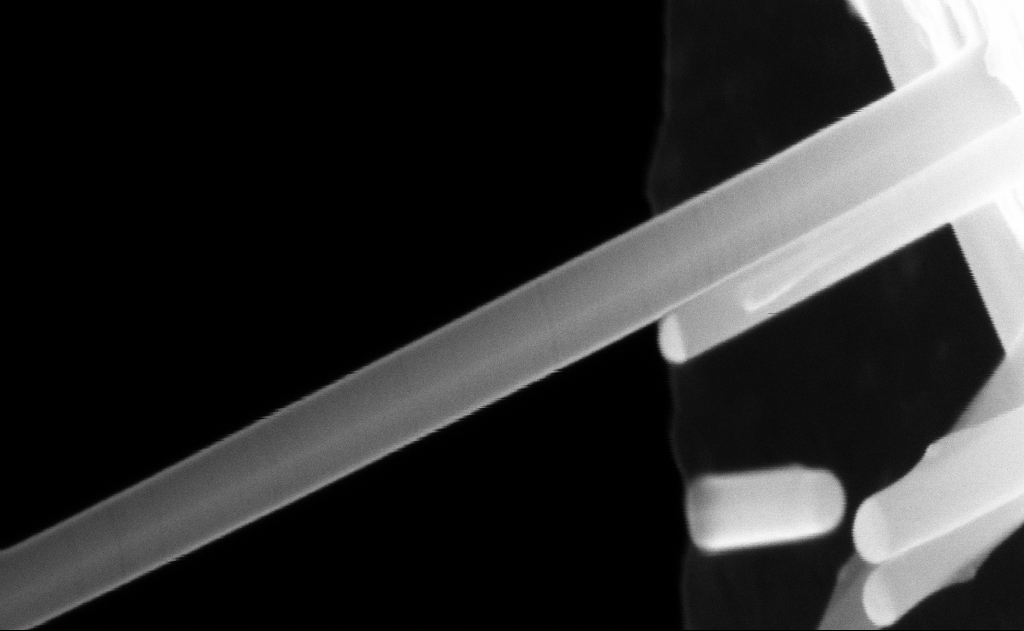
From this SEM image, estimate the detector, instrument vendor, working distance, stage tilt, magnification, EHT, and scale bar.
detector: InLens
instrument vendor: Zeiss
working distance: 9 mm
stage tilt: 0°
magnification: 289.19 K X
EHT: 20 kV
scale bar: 100 nm